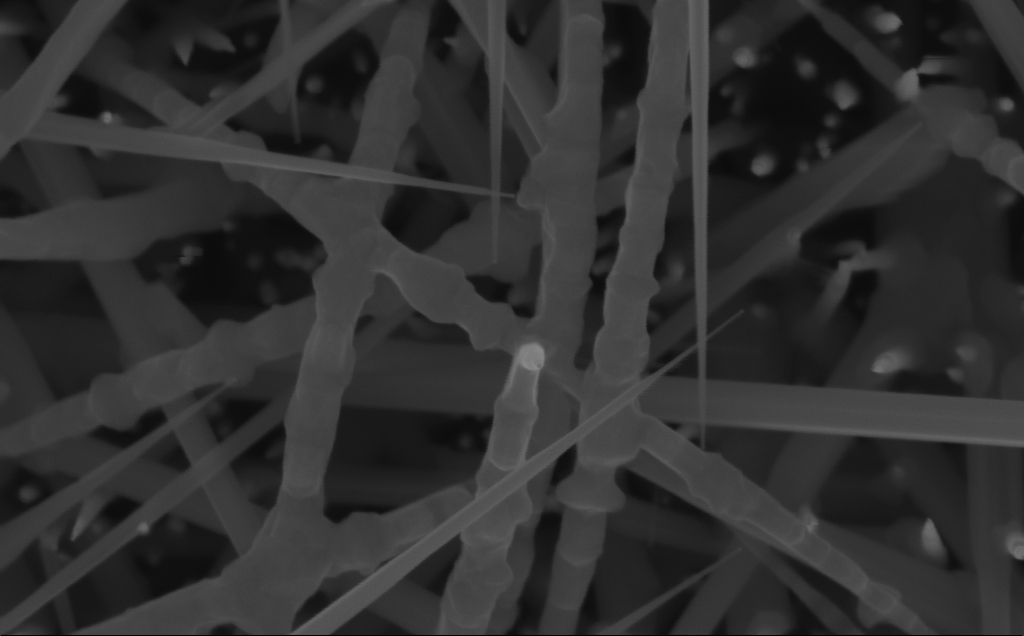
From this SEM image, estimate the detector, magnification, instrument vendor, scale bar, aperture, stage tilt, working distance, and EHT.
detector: InLens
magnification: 165.64 K X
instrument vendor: Zeiss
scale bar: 100 nm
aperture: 30 µm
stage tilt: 0°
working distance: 6 mm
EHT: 10 kV